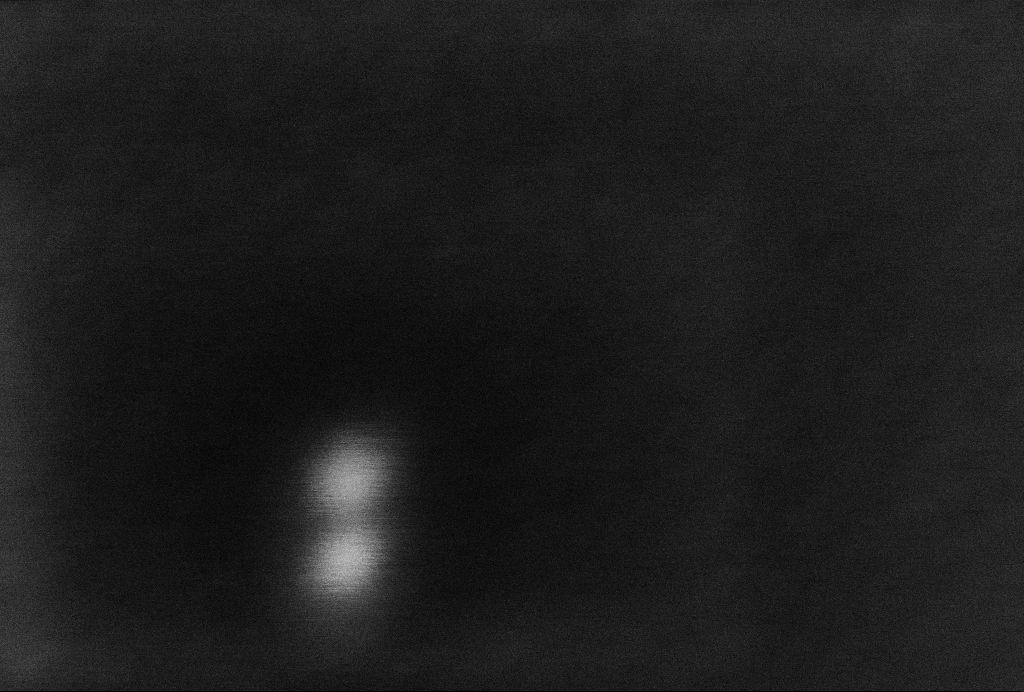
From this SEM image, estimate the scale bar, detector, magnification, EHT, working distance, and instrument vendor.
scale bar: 20 nm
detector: InLens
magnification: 1229.03 K X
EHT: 2 kV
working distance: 3.3 mm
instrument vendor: Zeiss